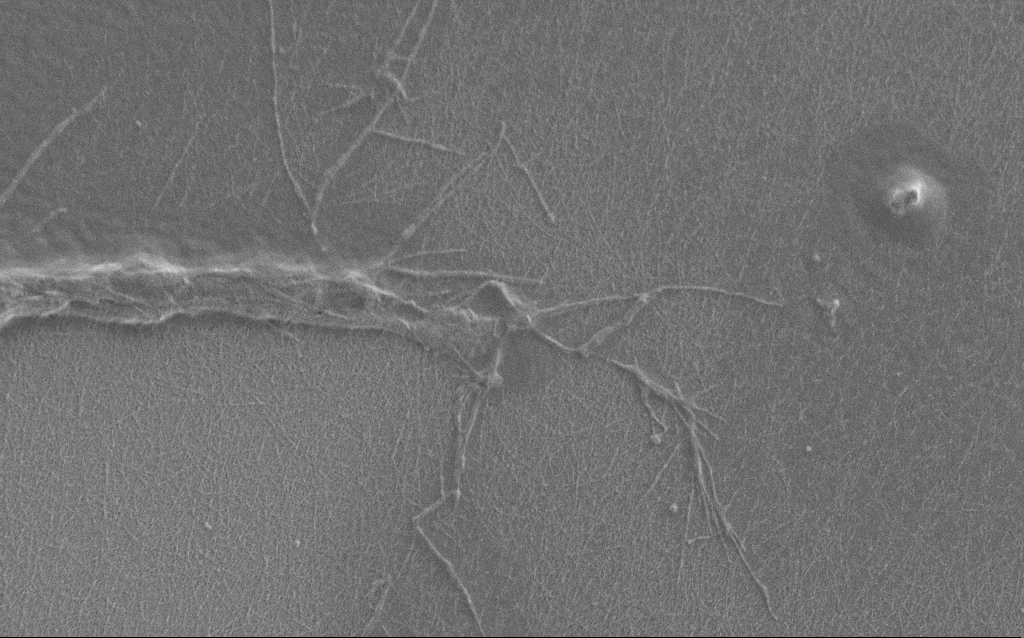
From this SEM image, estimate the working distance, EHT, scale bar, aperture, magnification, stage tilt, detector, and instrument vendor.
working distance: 6 mm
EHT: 1 kV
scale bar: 2000 nm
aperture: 30 µm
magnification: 7.5 K X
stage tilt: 0°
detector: SE2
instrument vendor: Zeiss